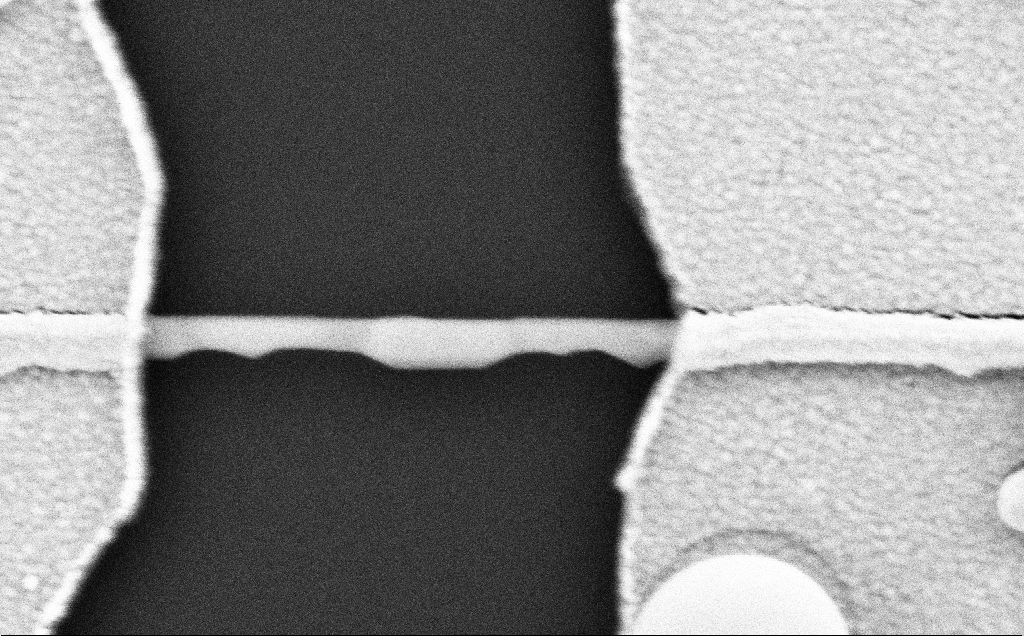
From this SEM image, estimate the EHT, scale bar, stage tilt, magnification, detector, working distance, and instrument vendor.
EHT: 5 kV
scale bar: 200 nm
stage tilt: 0°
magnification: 112.53 K X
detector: SE2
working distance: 10 mm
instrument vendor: Zeiss